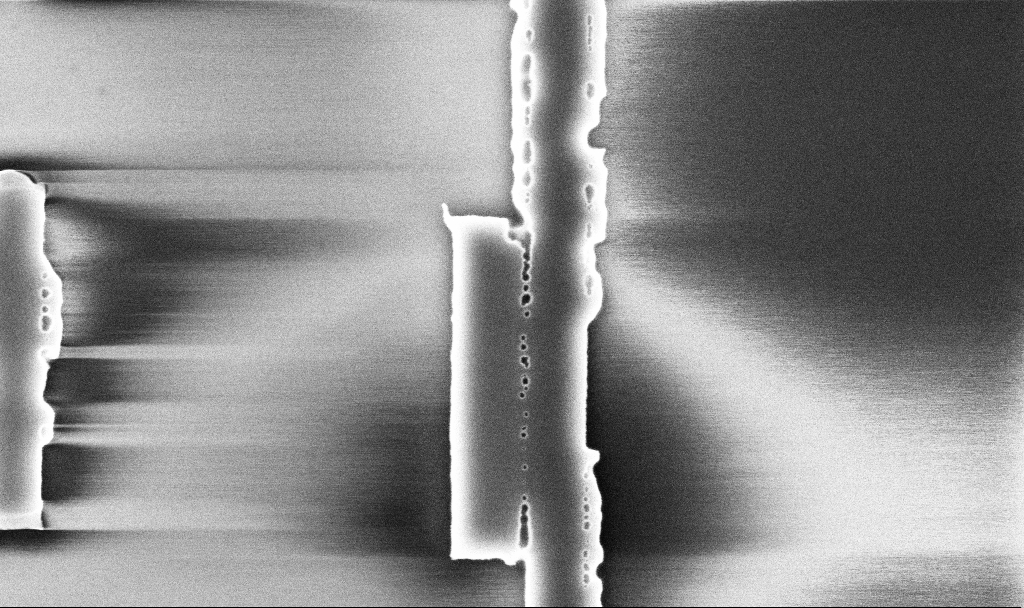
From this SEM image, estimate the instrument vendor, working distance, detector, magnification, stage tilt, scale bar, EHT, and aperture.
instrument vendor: Zeiss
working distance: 10.1 mm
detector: InLens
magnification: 42.49 K X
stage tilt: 0°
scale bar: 1000 nm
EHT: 5 kV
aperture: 30 µm